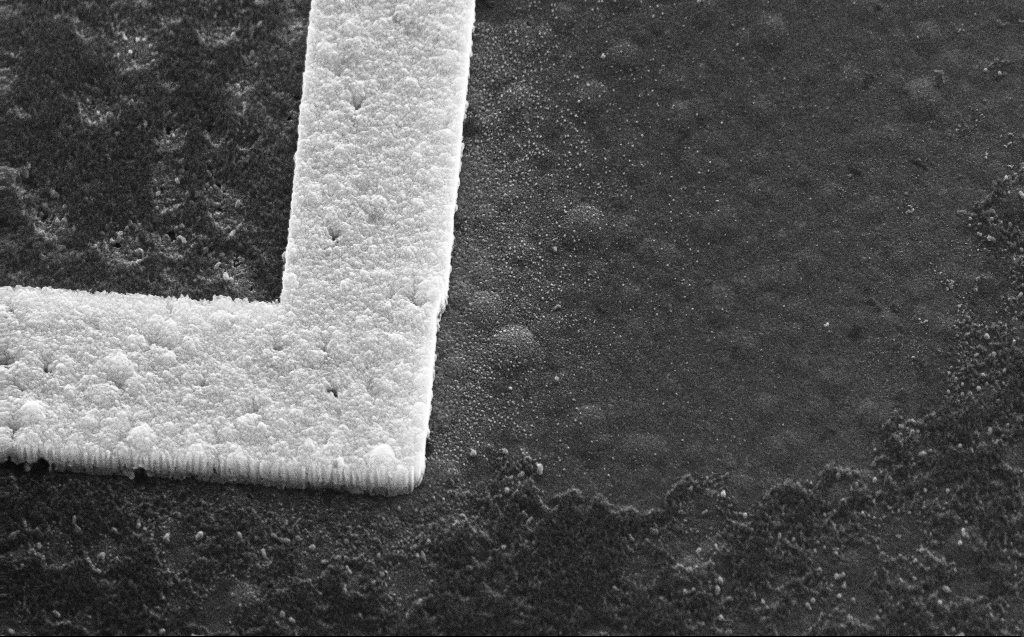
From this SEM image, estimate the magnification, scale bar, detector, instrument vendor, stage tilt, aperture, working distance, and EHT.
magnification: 61.76 K X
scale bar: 1000 nm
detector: SE2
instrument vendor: Zeiss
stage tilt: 45°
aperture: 30 µm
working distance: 5 mm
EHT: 30 kV